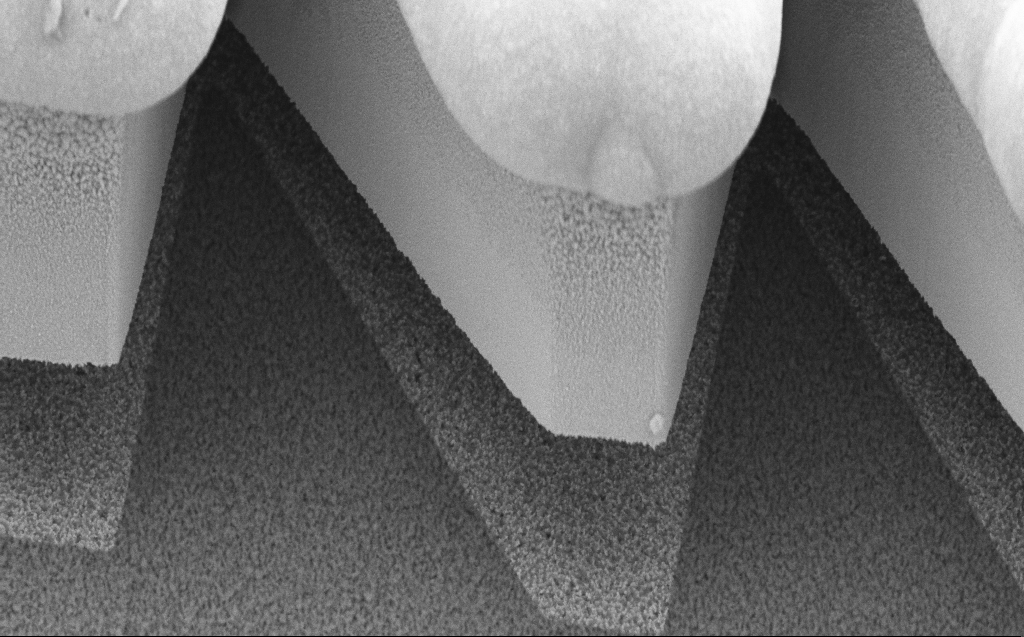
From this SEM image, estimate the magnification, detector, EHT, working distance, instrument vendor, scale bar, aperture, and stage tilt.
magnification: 17.8 K X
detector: SE2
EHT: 5 kV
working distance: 9 mm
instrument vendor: Zeiss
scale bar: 2000 nm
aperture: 30 µm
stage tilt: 45°